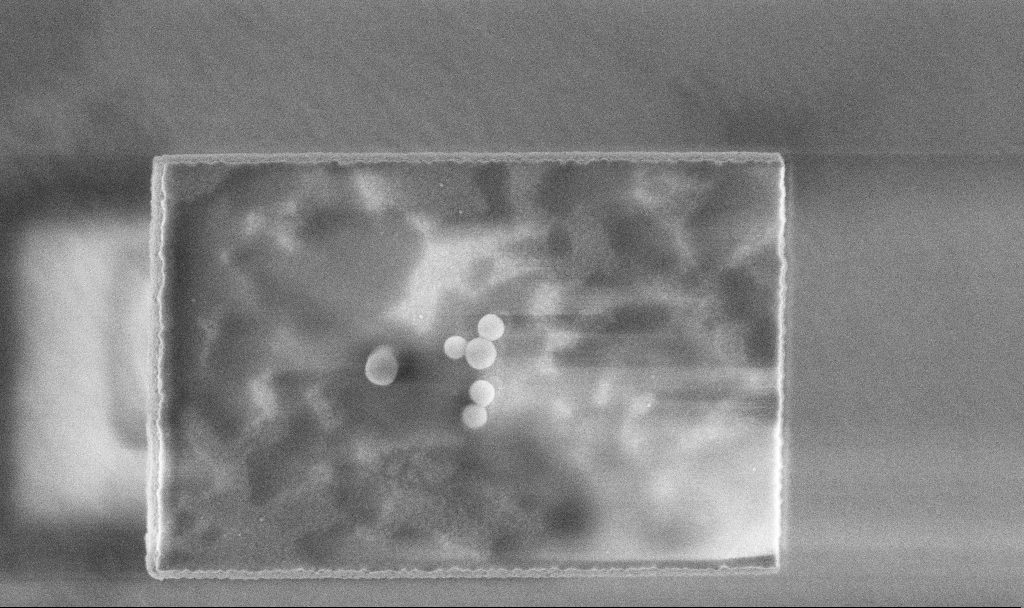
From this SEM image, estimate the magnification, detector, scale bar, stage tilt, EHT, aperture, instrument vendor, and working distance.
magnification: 51.2 K X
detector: InLens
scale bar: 1000 nm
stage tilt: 0°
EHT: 3 kV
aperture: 30 µm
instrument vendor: Zeiss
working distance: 3.3 mm